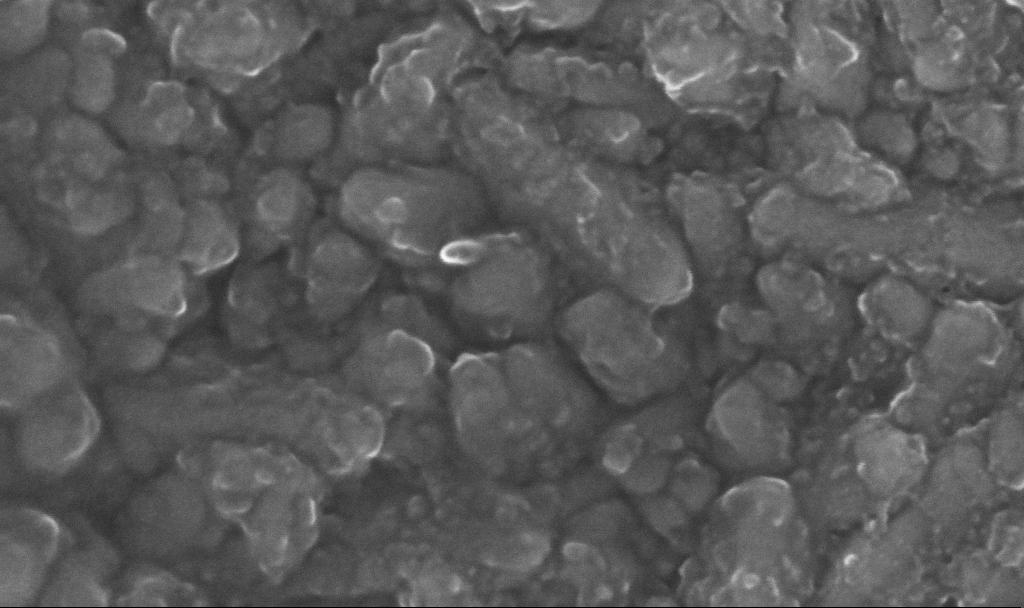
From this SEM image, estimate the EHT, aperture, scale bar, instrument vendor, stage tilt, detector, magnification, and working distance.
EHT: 20 kV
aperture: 30 µm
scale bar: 100 nm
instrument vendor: Zeiss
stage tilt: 0°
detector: InLens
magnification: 400.61 K X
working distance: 4.8 mm